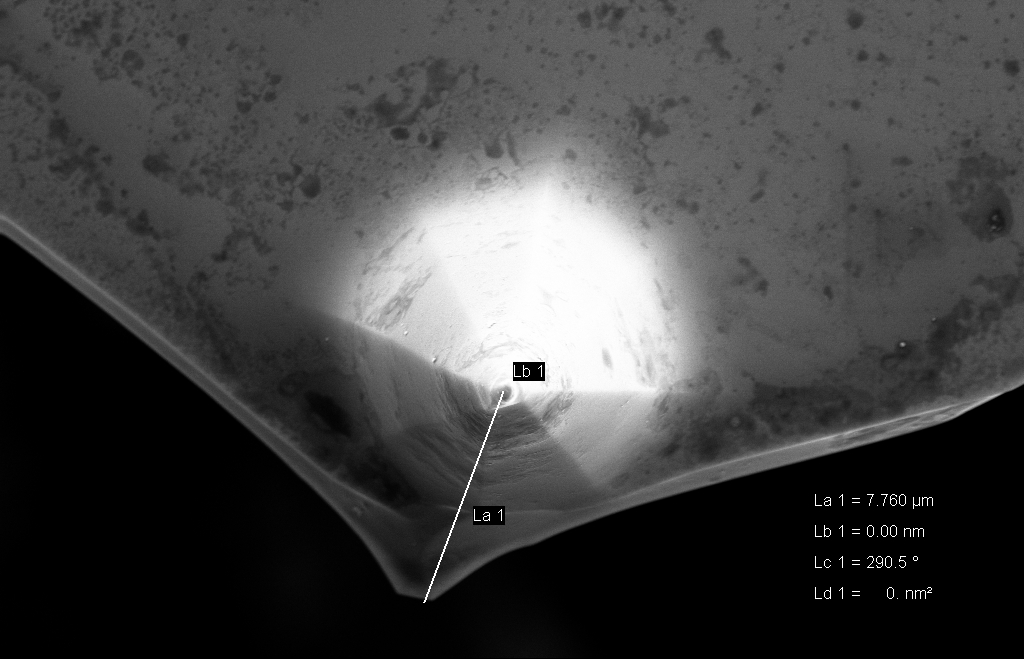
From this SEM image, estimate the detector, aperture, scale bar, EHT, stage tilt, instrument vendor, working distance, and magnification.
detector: InLens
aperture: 30 µm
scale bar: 2000 nm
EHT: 10 kV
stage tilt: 0°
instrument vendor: Zeiss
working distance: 8 mm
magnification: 10.66 K X